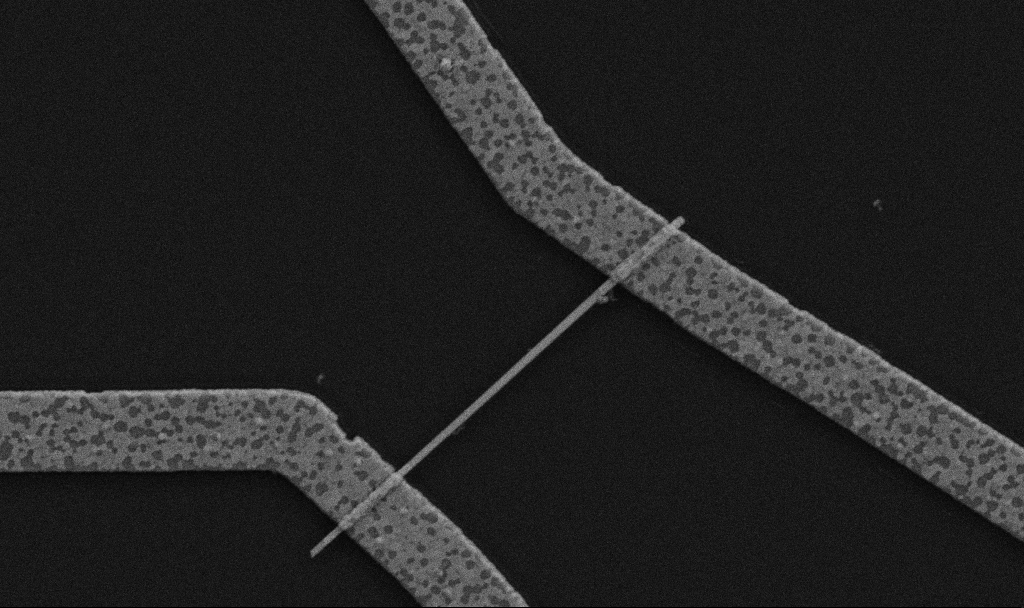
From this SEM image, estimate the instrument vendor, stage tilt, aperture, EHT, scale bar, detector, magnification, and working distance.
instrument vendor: Zeiss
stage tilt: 0°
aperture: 30 µm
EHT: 5 kV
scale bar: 1000 nm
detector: SE2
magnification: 30 K X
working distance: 10.7 mm